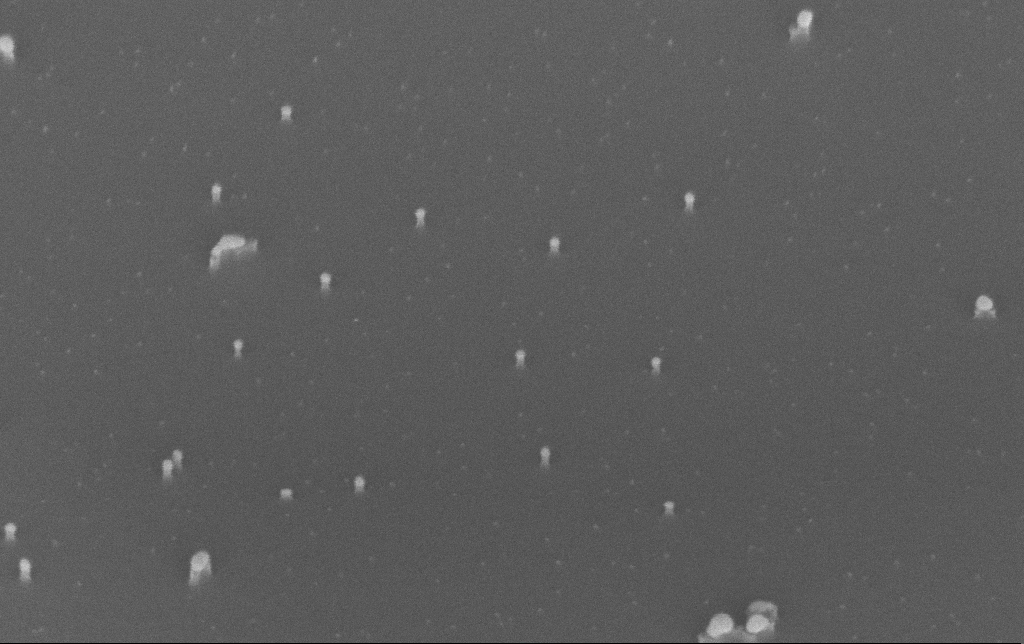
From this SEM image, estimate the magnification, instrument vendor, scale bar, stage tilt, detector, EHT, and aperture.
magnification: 200 K X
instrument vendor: Zeiss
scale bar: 100 nm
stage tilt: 45°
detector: InLens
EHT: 10 kV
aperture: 30 µm